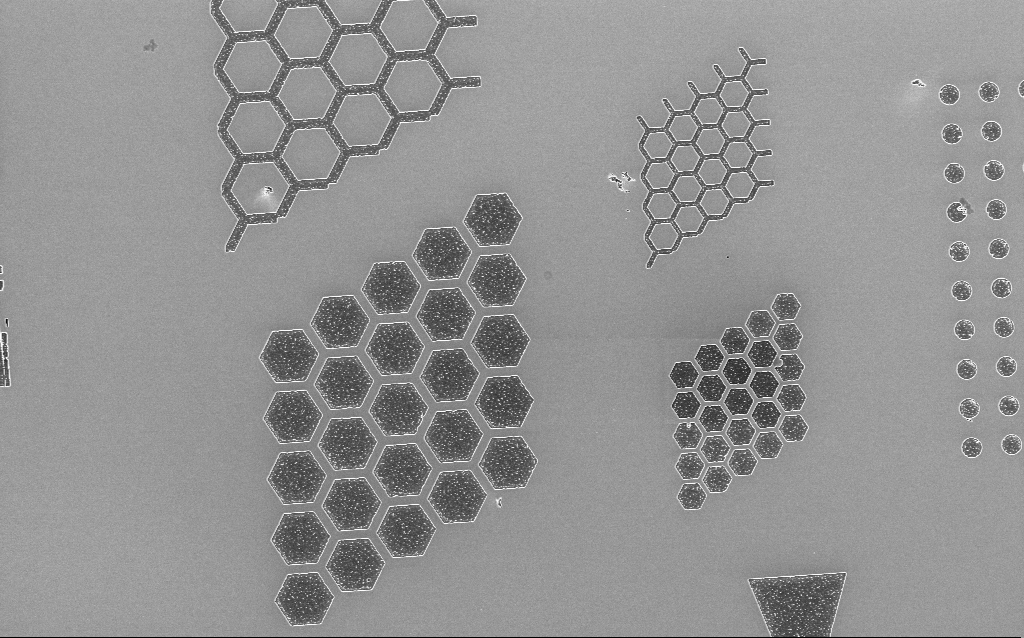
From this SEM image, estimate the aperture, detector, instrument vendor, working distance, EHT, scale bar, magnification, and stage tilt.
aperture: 30 µm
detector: InLens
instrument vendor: Zeiss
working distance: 5 mm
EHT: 2 kV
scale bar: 10000 nm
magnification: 3.63 K X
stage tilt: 0°